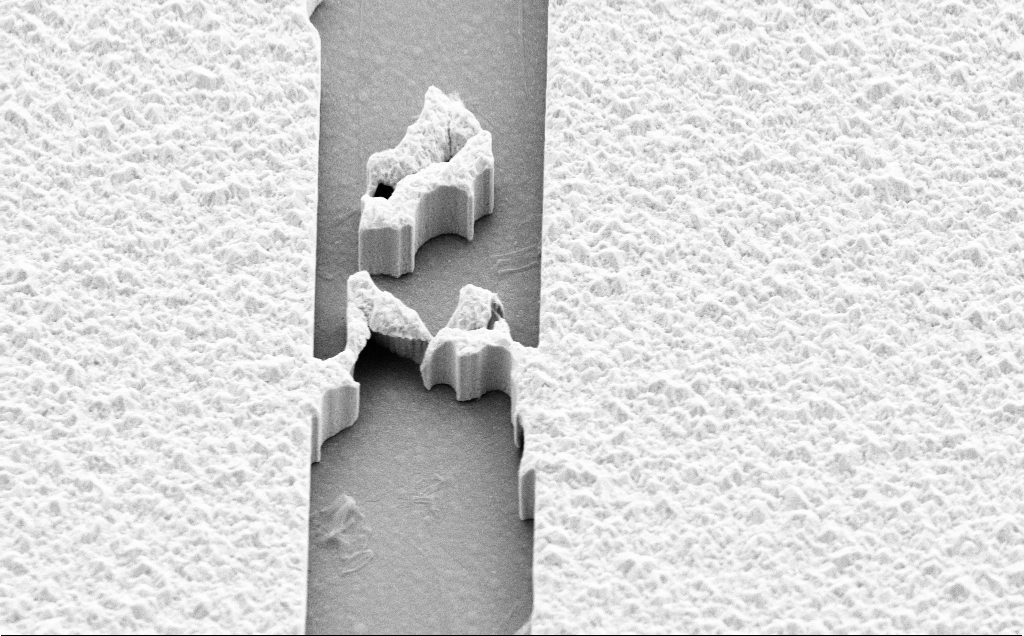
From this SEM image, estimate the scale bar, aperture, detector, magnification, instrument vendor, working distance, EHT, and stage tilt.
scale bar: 2000 nm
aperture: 30 µm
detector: SE2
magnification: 8.17 K X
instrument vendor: Zeiss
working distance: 8 mm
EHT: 10 kV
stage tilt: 45°